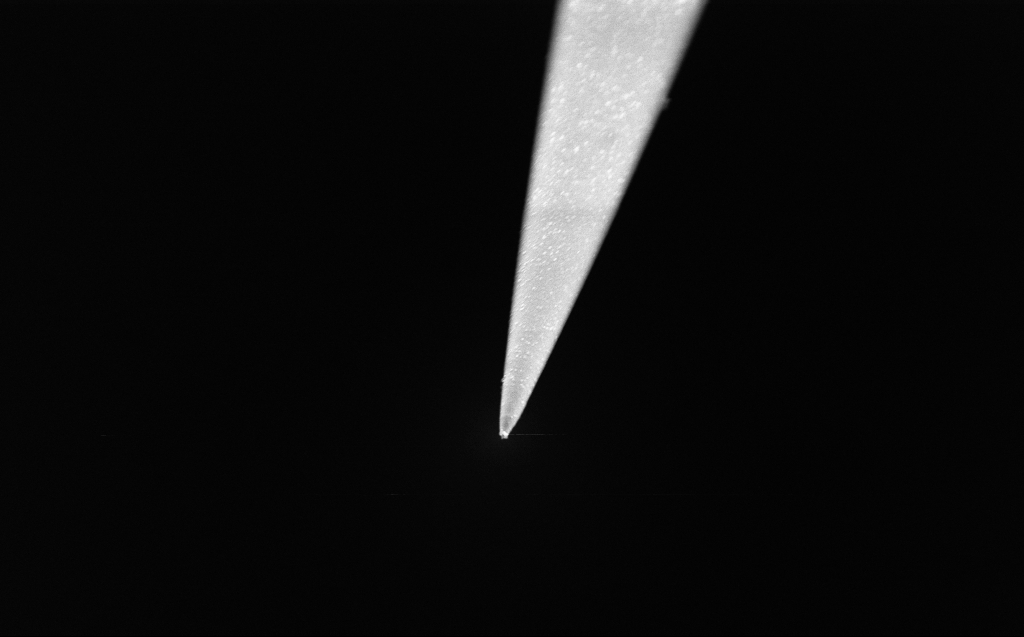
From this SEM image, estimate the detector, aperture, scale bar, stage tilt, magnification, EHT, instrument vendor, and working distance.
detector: InLens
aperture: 30 µm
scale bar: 1000 nm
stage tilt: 45°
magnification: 26.7 K X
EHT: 2.5 kV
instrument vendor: Zeiss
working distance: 3 mm